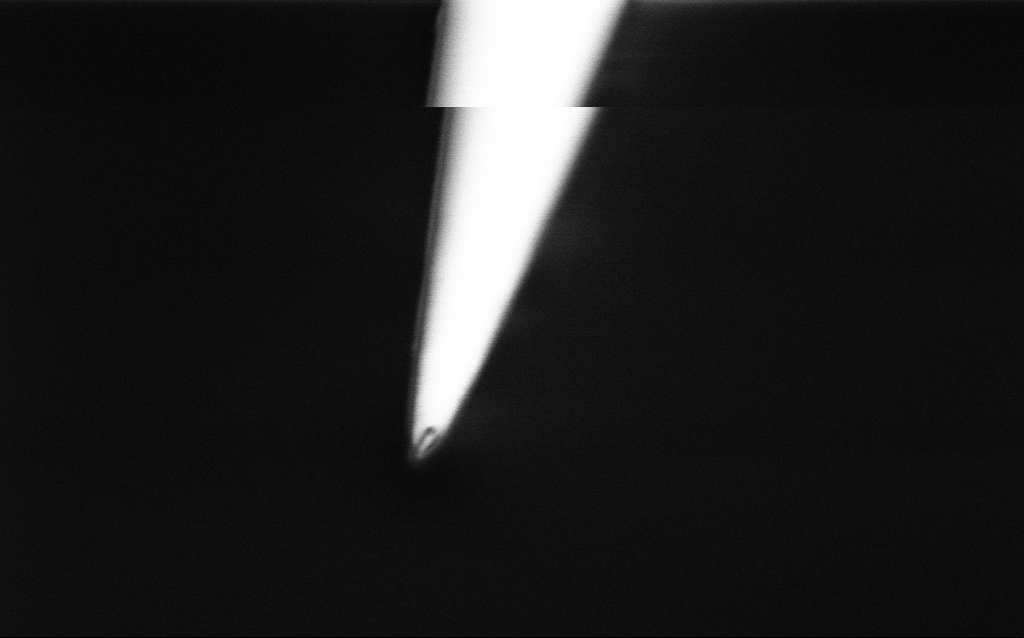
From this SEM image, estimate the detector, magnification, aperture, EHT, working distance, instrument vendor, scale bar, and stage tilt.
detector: InLens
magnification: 100 K X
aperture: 30 µm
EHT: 1 kV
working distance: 6.9 mm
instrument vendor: Zeiss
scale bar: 200 nm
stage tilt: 45°